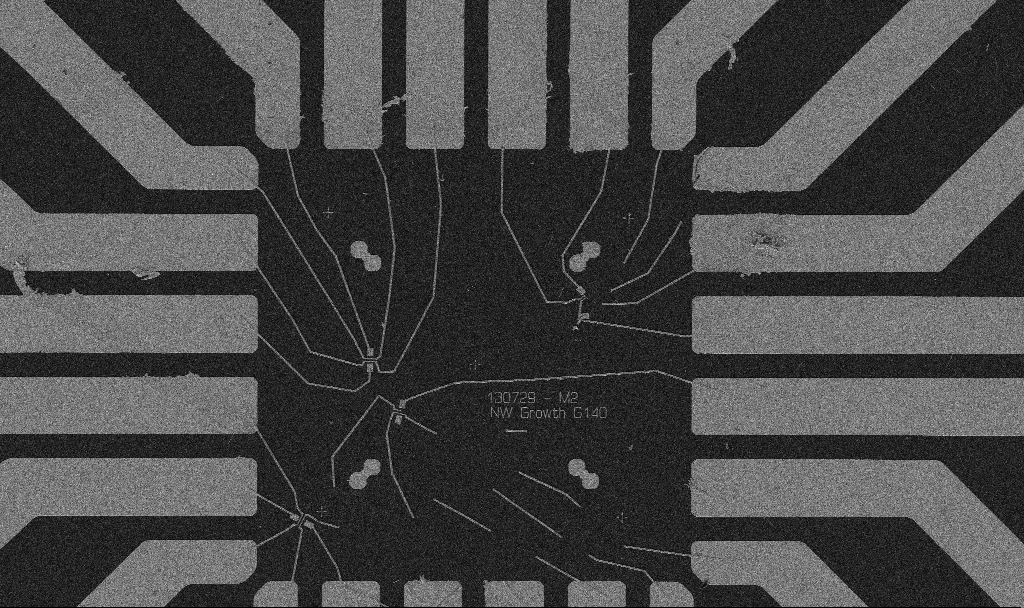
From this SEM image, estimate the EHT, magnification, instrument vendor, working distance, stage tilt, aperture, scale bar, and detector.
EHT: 5 kV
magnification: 1 K X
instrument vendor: Zeiss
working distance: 10.7 mm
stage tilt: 0°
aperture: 30 µm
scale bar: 20000 nm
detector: SE2